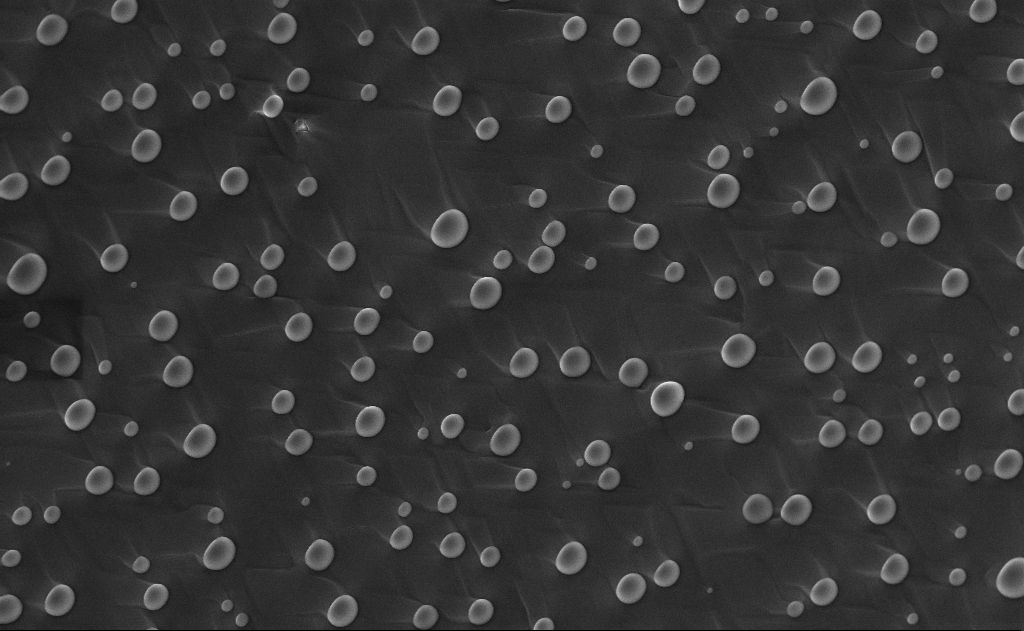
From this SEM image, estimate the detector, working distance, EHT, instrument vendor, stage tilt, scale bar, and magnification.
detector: InLens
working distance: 11 mm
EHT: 10 kV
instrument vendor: Zeiss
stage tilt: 0°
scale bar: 2000 nm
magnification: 10 K X